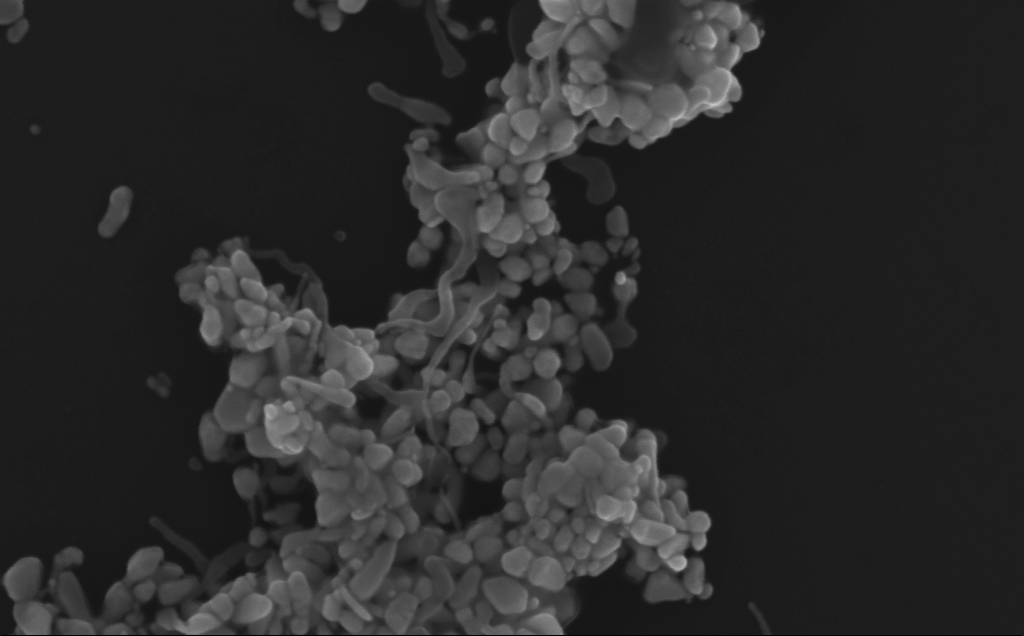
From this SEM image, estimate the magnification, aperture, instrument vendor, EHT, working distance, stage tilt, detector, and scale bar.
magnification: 165.96 K X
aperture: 30 µm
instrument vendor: Zeiss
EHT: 10 kV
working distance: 3 mm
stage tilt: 0°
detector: InLens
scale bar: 200 nm